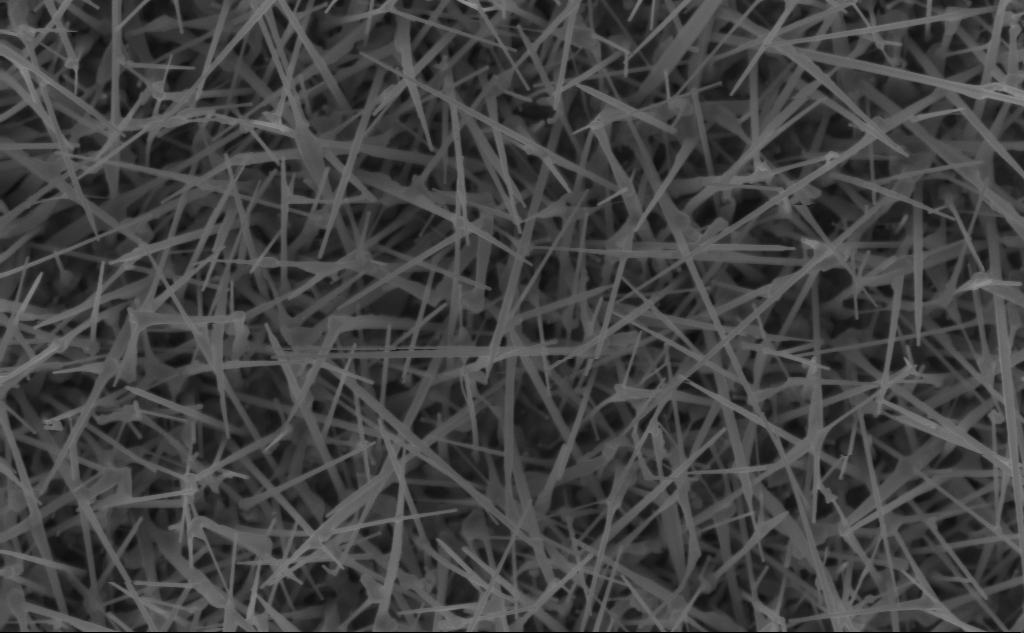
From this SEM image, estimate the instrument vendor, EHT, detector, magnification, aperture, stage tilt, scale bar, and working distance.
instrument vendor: Zeiss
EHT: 5 kV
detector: InLens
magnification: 40 K X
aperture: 30 µm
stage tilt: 0°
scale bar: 1000 nm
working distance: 4 mm